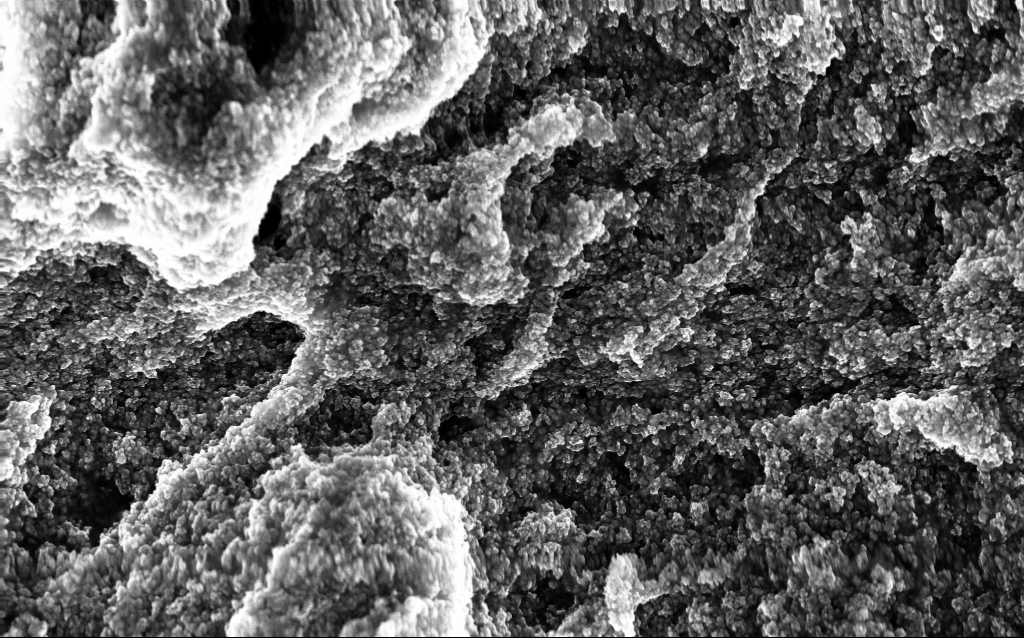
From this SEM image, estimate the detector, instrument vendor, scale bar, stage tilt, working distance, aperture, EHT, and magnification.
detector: InLens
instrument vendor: Zeiss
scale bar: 1000 nm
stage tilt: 0°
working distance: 2.6 mm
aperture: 30 µm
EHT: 10 kV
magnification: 37.88 K X